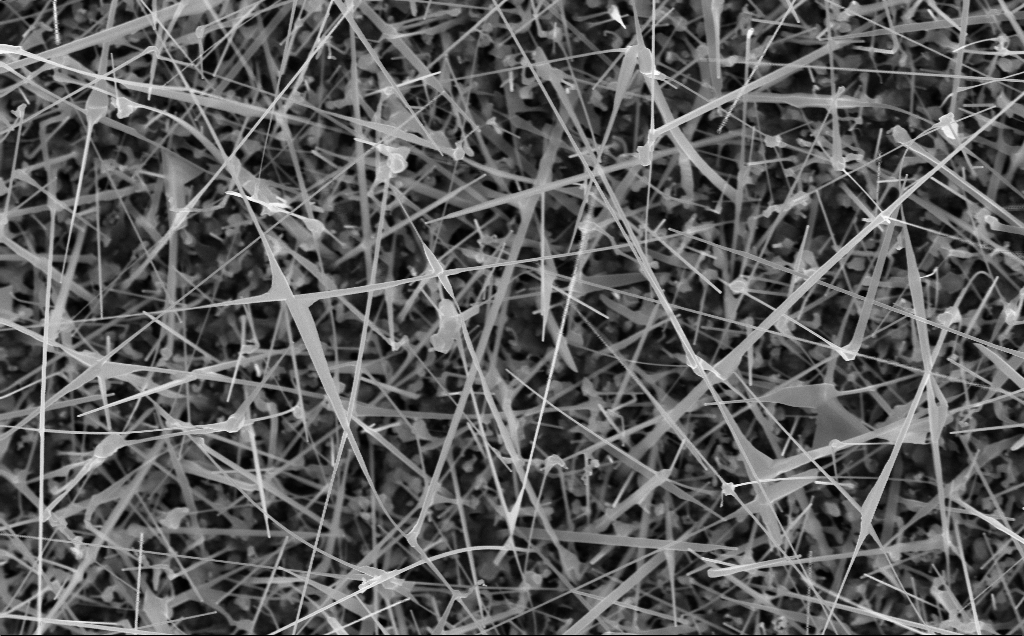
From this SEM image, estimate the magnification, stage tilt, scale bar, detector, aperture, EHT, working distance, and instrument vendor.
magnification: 30 K X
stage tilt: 0°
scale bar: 2000 nm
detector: InLens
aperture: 30 µm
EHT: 10 kV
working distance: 8 mm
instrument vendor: Zeiss